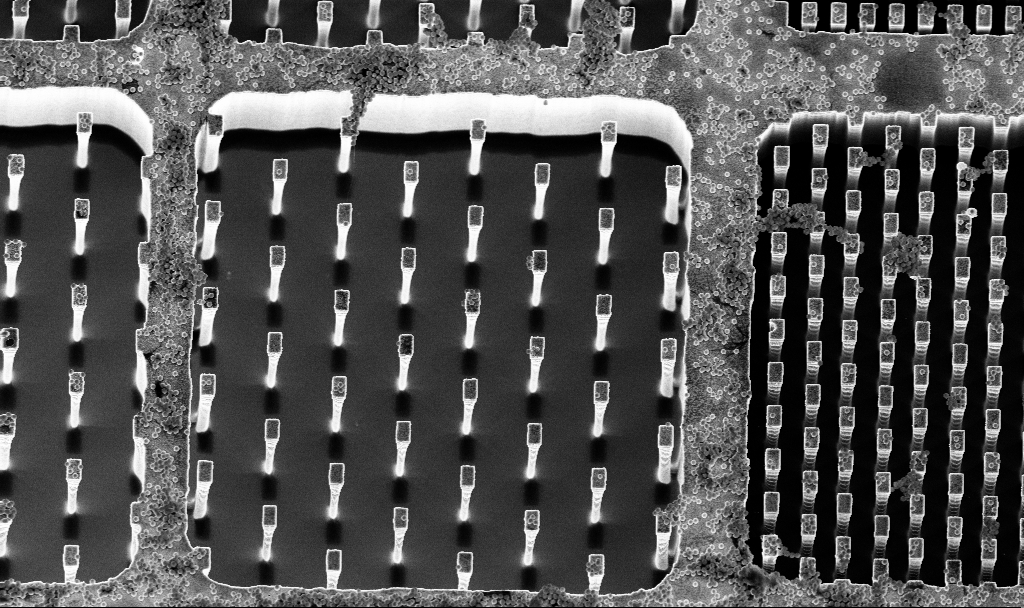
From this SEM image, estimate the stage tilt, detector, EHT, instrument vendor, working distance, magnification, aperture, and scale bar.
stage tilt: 15°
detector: InLens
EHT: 5 kV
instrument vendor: Zeiss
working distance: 3.2 mm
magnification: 3.39 K X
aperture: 30 µm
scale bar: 10000 nm